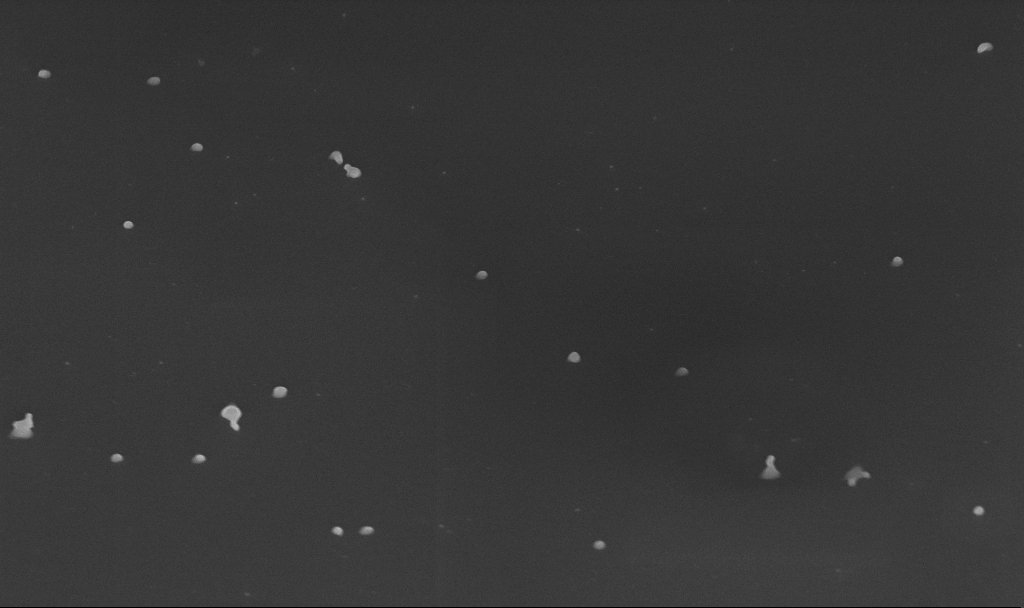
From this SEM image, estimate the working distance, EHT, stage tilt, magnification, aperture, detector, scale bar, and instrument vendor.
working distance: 5.6 mm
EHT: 10 kV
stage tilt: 45°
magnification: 50 K X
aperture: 30 µm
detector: InLens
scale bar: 1000 nm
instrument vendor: Zeiss